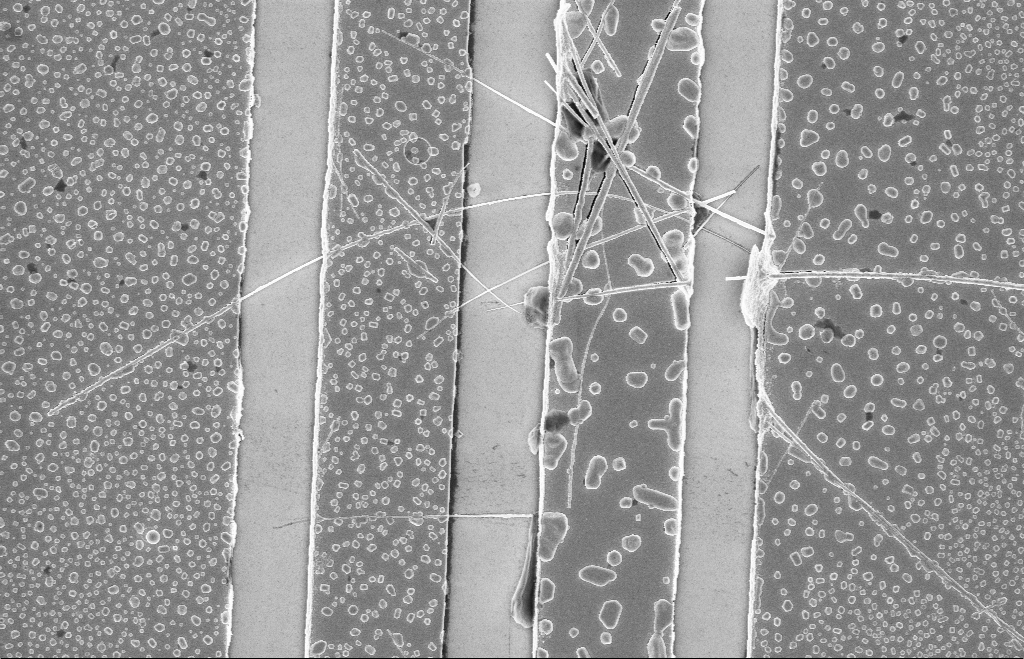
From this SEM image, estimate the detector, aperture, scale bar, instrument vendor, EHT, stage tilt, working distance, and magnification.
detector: InLens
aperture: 20 µm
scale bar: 2000 nm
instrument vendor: Zeiss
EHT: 5 kV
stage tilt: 0°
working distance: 8 mm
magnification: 13.63 K X